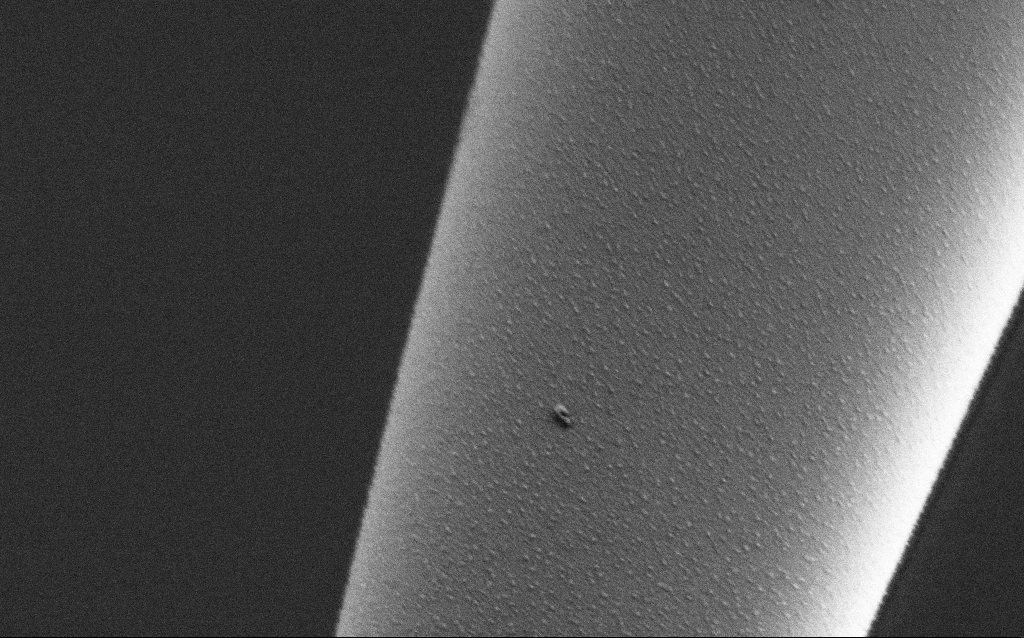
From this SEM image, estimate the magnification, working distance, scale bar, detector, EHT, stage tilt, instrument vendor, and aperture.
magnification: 50 K X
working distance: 6.5 mm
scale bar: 1000 nm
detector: SE2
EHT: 1 kV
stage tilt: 45°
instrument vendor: Zeiss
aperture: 30 µm